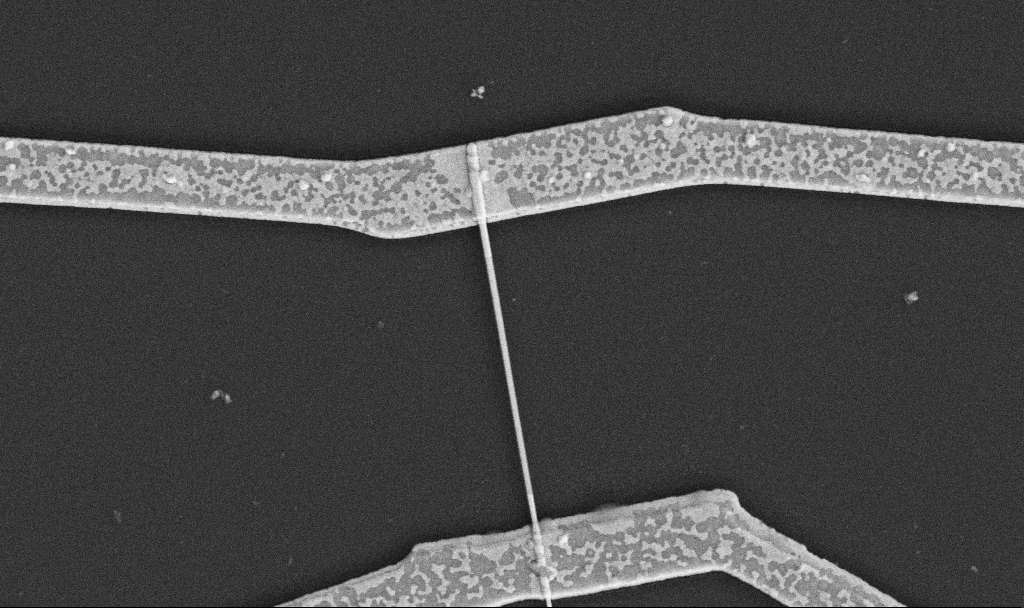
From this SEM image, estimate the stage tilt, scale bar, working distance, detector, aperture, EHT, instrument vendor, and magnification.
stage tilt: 0°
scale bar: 1000 nm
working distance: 10.6 mm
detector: SE2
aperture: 30 µm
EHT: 5 kV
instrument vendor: Zeiss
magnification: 30 K X